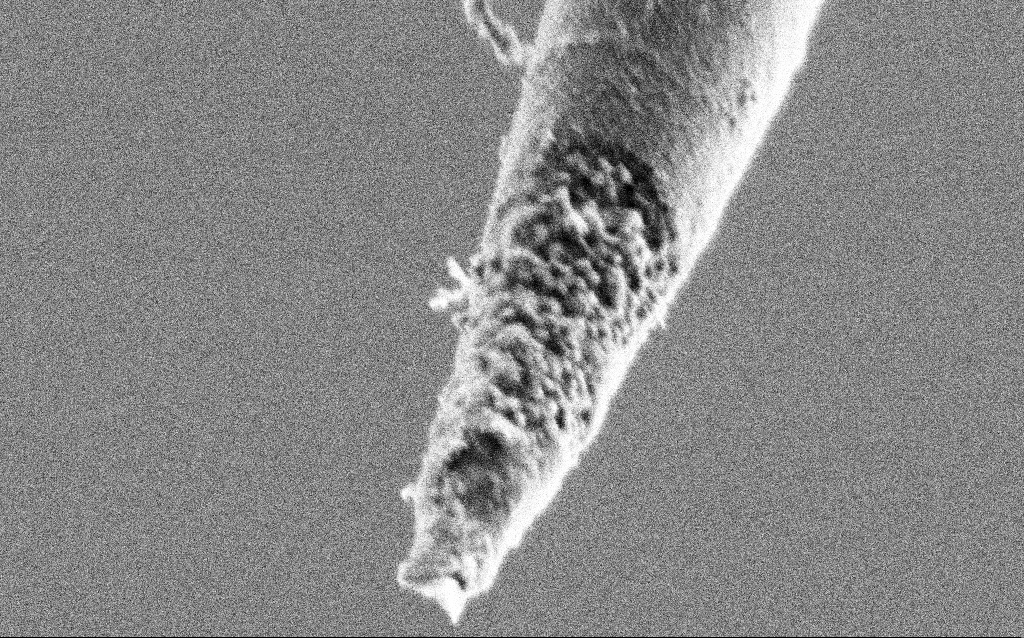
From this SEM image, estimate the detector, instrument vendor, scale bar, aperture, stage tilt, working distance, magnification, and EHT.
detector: SE2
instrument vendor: Zeiss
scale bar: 200 nm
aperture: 30 µm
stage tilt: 45°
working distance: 6.5 mm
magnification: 100 K X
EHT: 1 kV